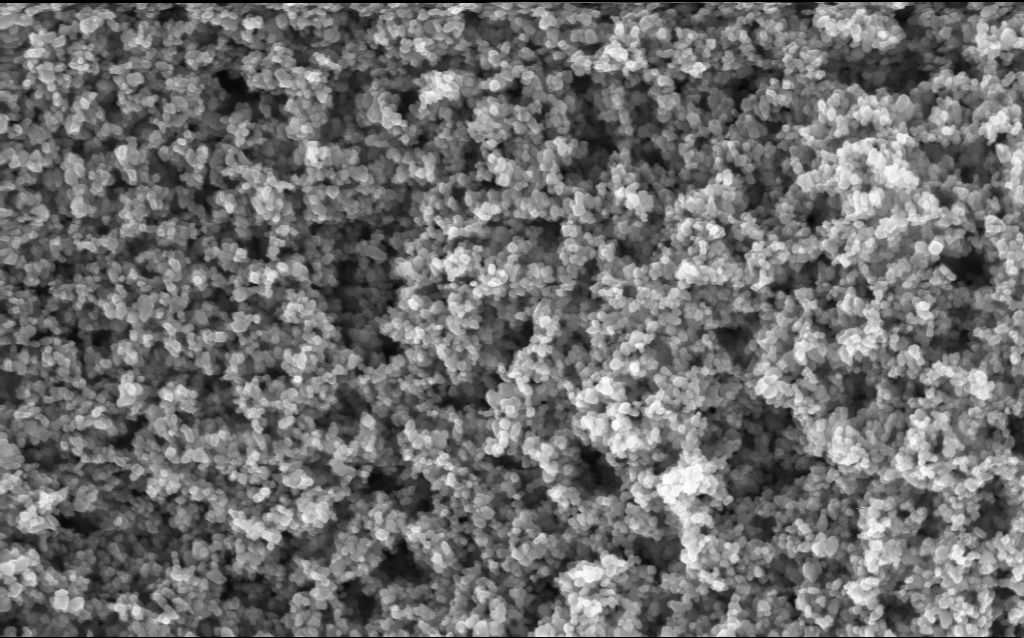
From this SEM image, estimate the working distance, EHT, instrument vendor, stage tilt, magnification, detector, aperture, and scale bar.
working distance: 2.9 mm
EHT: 5 kV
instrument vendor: Zeiss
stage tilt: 0°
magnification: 130 K X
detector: InLens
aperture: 30 µm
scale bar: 100 nm